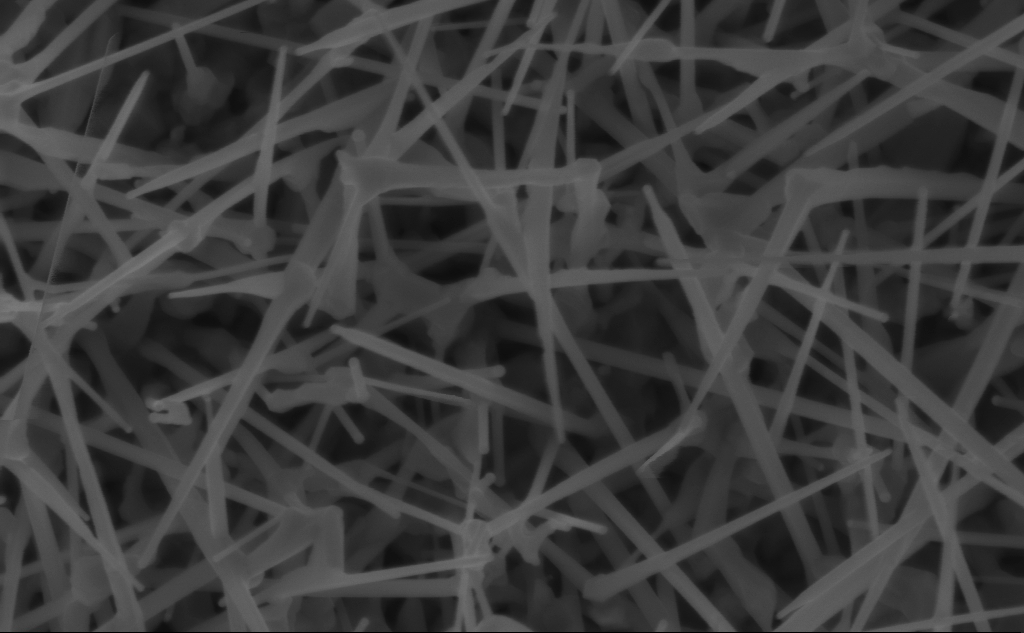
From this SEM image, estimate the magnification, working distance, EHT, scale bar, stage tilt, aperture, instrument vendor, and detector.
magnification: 89.69 K X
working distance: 4 mm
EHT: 5 kV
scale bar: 200 nm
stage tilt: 0°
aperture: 30 µm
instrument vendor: Zeiss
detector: InLens